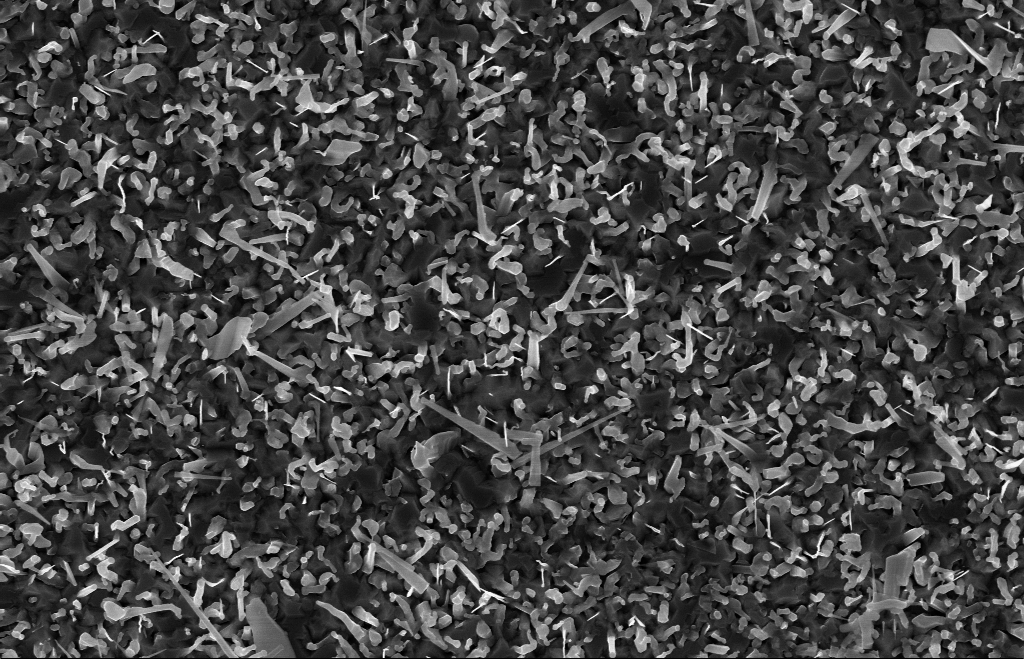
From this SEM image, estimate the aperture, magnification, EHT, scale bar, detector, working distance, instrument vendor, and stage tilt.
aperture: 30 µm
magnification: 20 K X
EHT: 10 kV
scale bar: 1000 nm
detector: InLens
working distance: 9 mm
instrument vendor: Zeiss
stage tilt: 0°